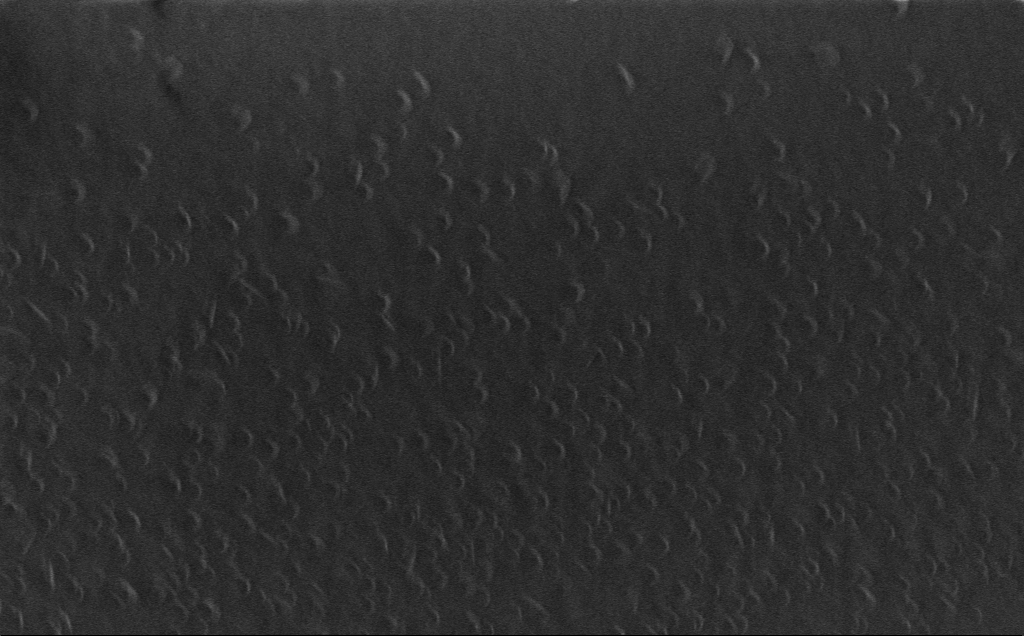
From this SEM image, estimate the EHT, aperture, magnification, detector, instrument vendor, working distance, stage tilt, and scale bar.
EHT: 1 kV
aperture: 30 µm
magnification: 14.53 K X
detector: InLens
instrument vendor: Zeiss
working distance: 3 mm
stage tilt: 0°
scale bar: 2000 nm